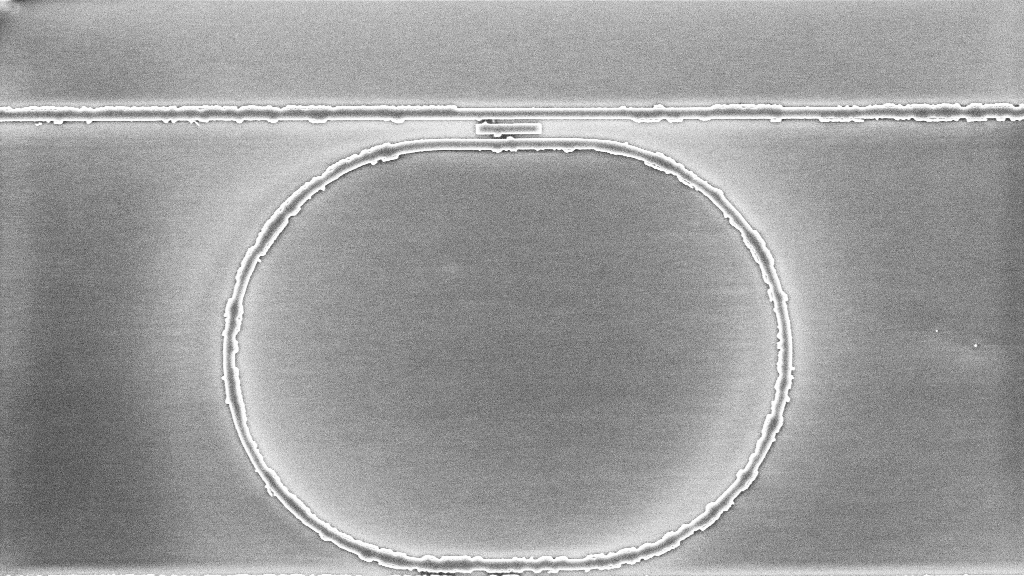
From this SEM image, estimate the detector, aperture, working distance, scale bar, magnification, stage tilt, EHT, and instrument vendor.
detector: InLens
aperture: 30 µm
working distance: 10.1 mm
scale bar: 2000 nm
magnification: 8.05 K X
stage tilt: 0°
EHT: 5 kV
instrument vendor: Zeiss